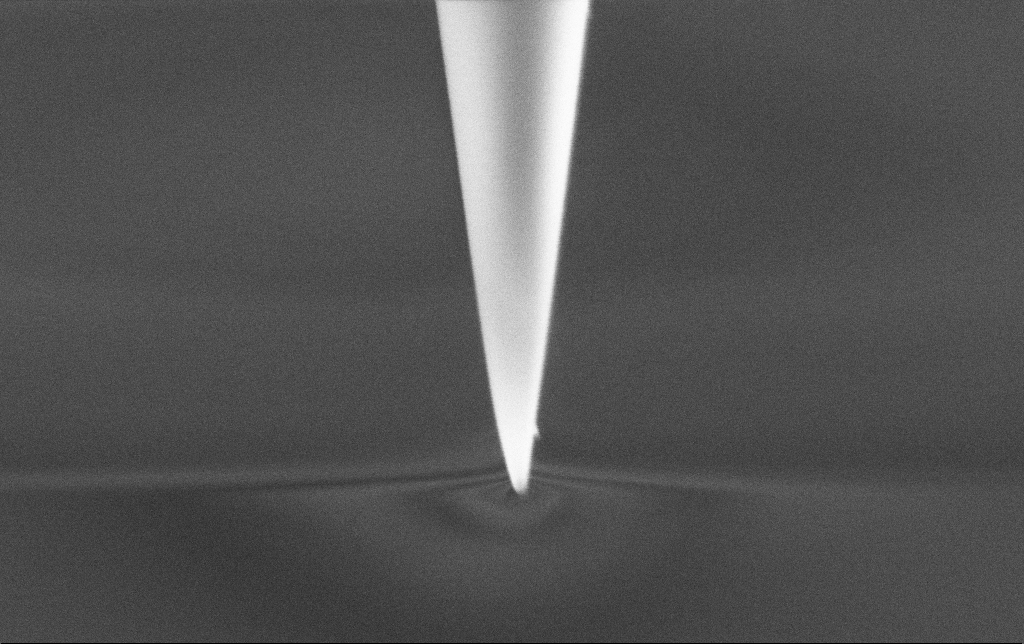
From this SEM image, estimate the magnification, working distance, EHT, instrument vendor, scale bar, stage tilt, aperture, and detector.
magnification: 75 K X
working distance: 7.6 mm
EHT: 2 kV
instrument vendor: Zeiss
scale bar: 200 nm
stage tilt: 45°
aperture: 30 µm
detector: SE2